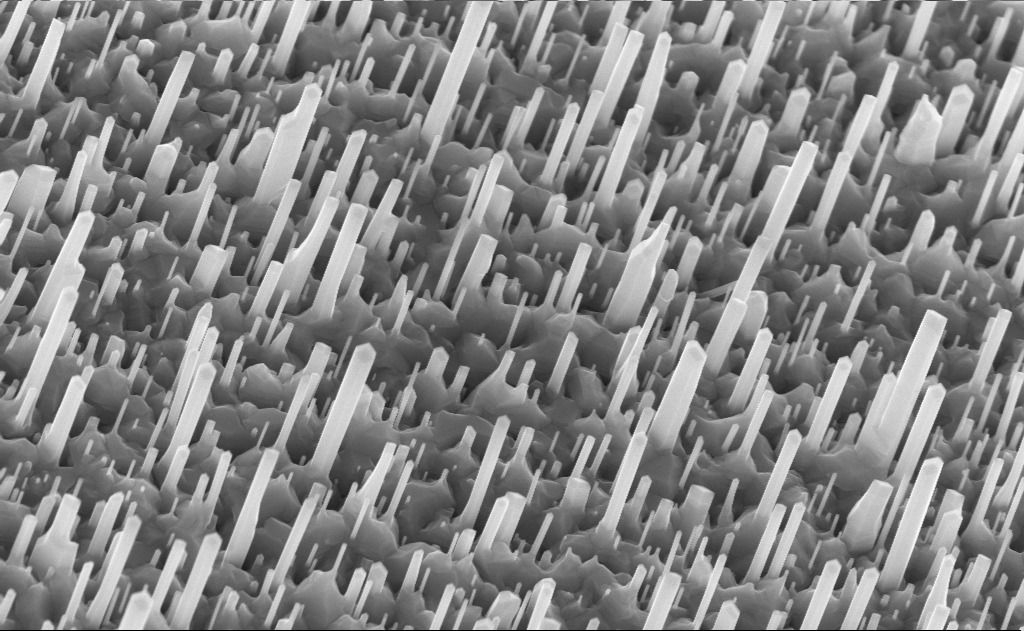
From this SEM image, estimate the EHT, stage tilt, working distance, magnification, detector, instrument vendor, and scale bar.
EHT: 10 kV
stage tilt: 0°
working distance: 9 mm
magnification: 40 K X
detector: InLens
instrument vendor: Zeiss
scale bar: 1000 nm